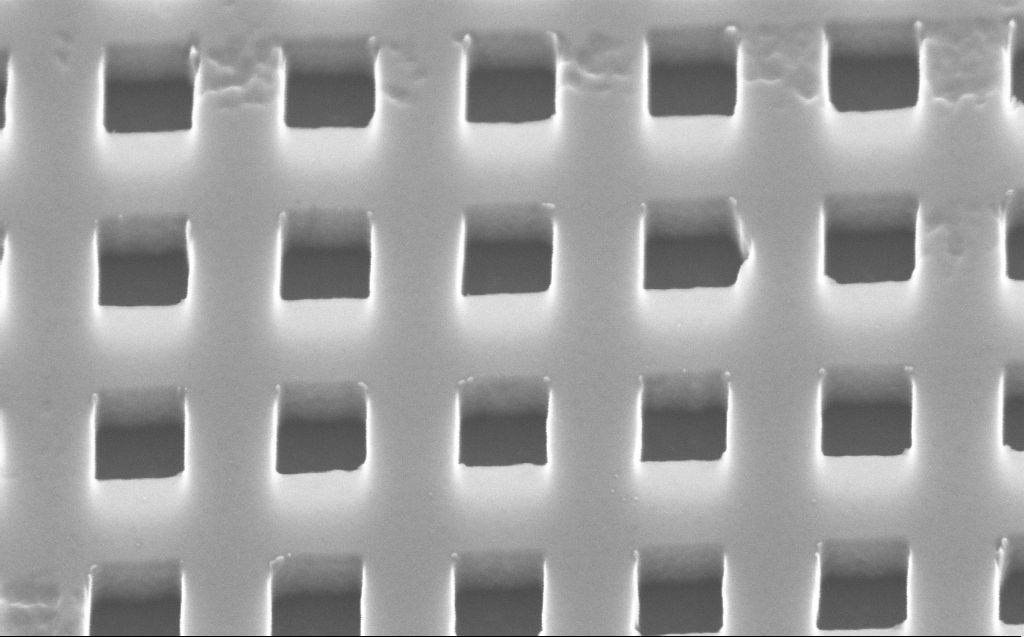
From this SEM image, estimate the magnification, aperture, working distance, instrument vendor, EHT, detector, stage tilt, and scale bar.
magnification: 135 K X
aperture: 30 µm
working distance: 3 mm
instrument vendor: Zeiss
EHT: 10 kV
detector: InLens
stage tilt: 45°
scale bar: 200 nm